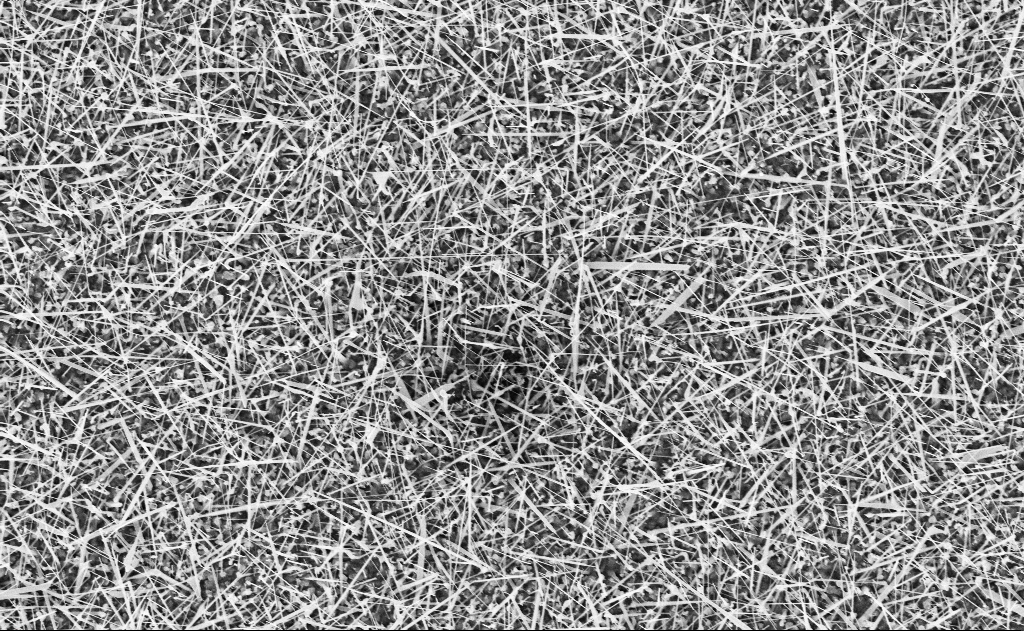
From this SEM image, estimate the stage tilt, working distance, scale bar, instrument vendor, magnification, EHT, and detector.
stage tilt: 0°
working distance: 20 mm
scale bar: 2000 nm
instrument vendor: Zeiss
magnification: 10 K X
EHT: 10 kV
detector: InLens